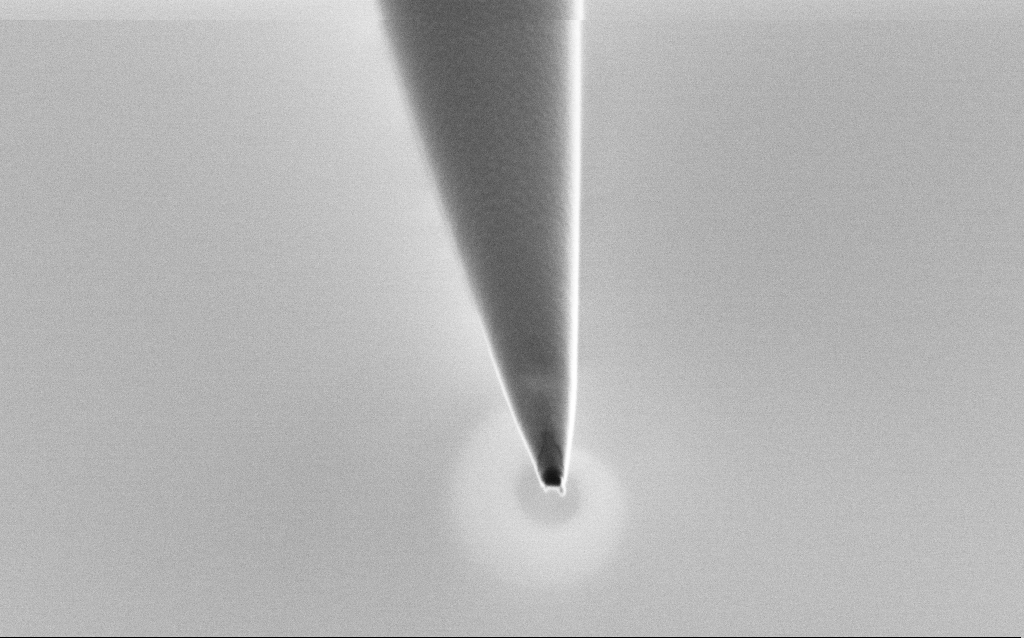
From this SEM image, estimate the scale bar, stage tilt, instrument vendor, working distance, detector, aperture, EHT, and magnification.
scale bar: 1000 nm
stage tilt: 45°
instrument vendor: Zeiss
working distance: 6 mm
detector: SE2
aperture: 30 µm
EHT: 1 kV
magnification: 50 K X